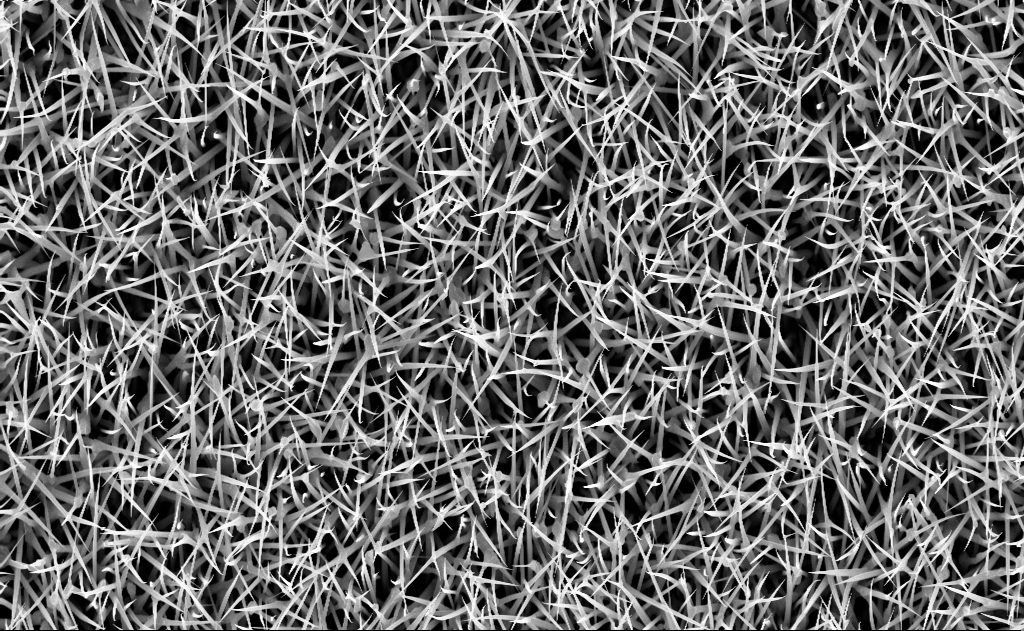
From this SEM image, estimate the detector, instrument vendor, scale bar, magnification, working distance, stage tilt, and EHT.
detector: InLens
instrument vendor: Zeiss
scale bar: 2000 nm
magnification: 10 K X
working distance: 7 mm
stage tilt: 0°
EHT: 10 kV